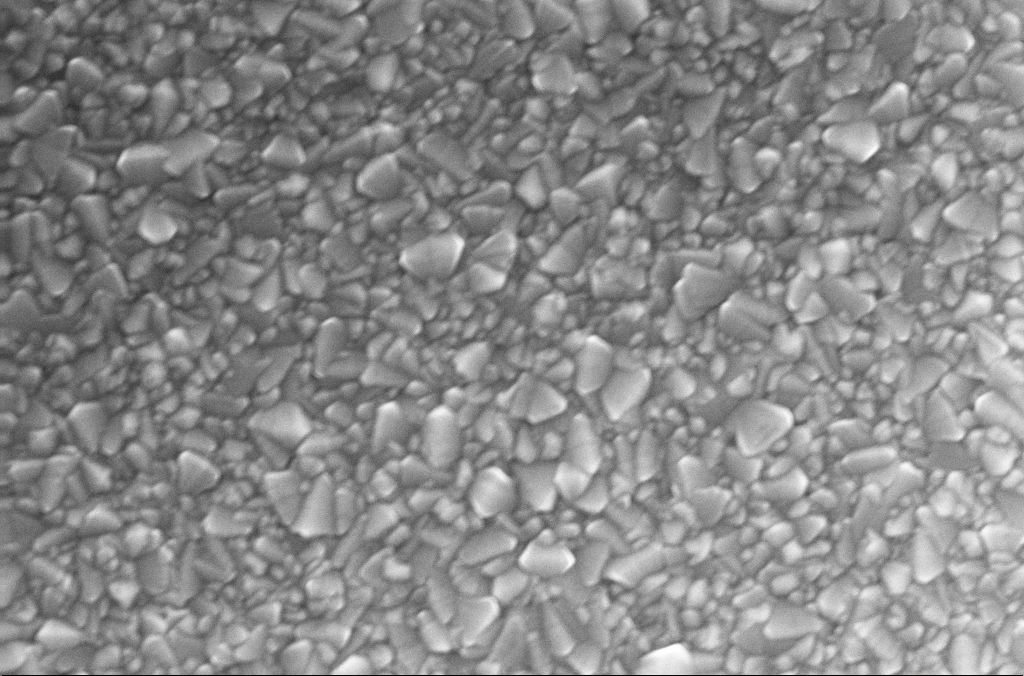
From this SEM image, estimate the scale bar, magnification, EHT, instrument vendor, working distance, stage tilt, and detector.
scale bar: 1000 nm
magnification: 50 K X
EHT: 20 kV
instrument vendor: Zeiss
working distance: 2 mm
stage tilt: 0°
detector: InLens